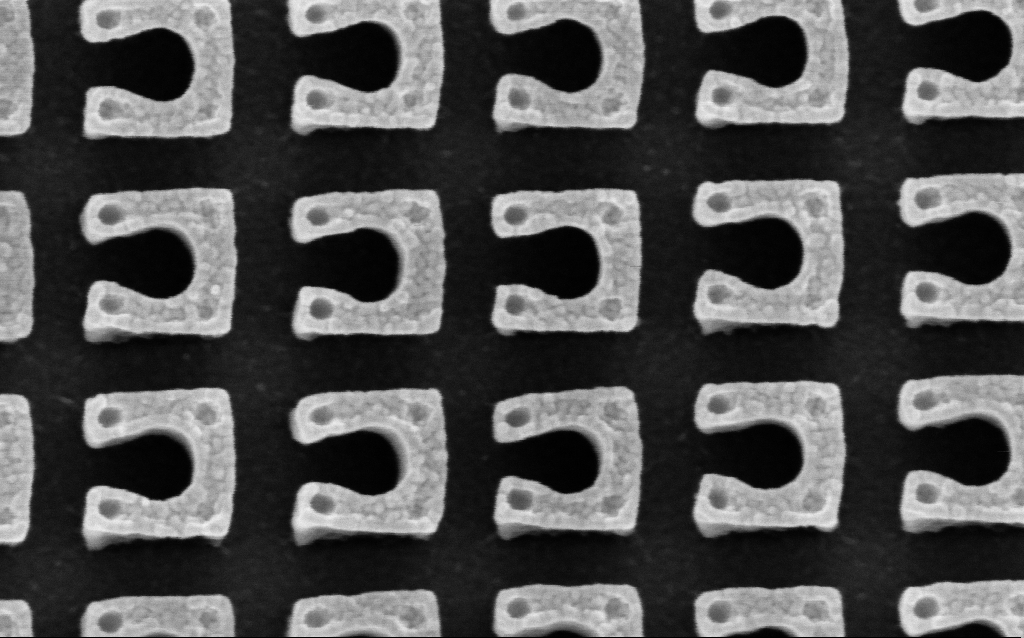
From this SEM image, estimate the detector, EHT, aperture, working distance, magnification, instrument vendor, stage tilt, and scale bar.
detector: InLens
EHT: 3 kV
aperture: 30 µm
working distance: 4.6 mm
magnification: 163.84 K X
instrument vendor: Zeiss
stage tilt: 0°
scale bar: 200 nm